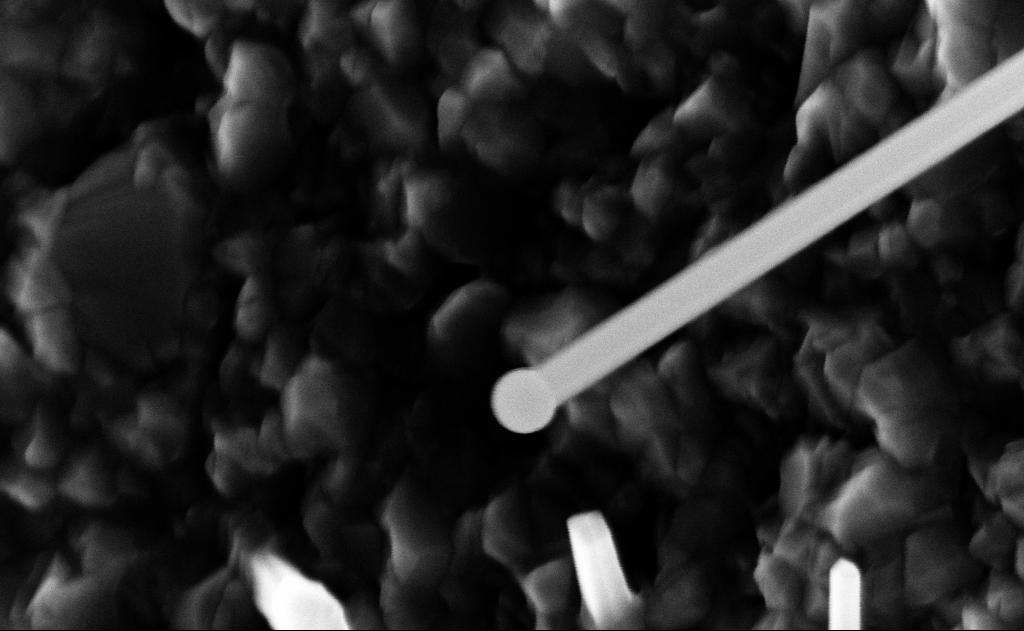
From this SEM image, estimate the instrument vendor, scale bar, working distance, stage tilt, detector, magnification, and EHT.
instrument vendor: Zeiss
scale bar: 200 nm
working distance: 9 mm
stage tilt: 0°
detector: InLens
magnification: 150 K X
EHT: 10 kV